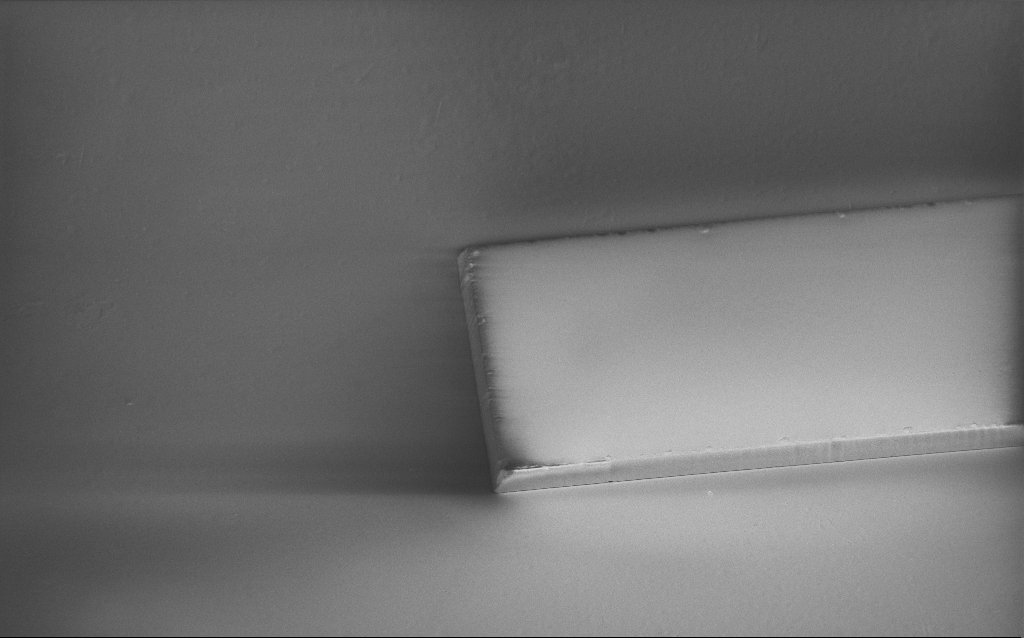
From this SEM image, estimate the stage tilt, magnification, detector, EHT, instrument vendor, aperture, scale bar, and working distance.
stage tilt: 45°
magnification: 1.46 K X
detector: InLens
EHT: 1.2 kV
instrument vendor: Zeiss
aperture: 30 µm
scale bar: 20000 nm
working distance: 6 mm